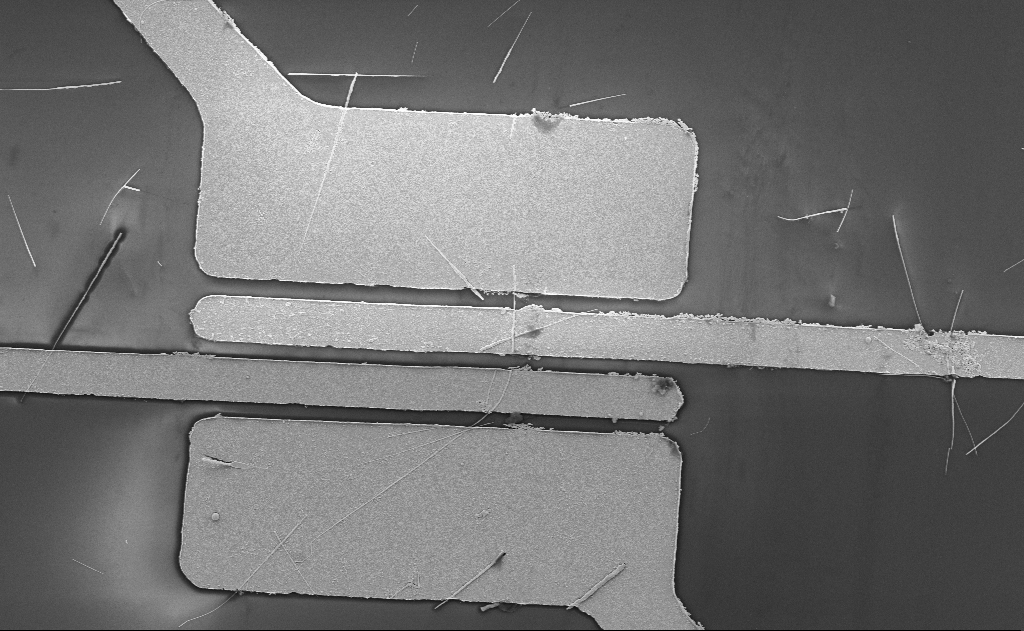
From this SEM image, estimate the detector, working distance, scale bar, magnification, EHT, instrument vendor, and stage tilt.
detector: SE2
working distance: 15 mm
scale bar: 10000 nm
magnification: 5.83 K X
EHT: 5 kV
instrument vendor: Zeiss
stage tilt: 0°